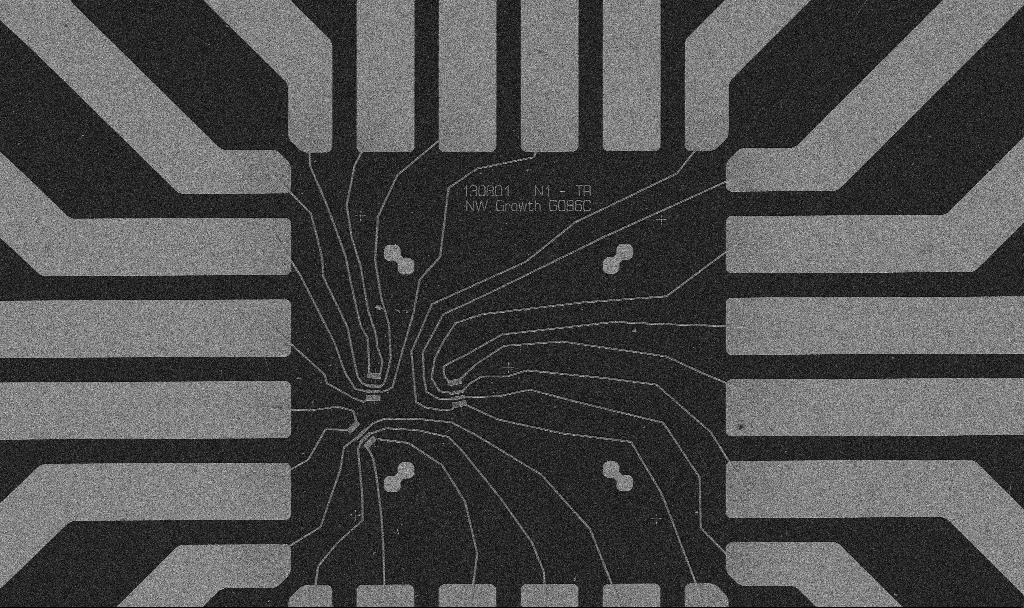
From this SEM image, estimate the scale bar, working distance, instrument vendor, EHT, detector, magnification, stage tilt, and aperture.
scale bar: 20000 nm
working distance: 10.7 mm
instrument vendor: Zeiss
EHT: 5 kV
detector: SE2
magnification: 1 K X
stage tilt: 0°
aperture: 30 µm